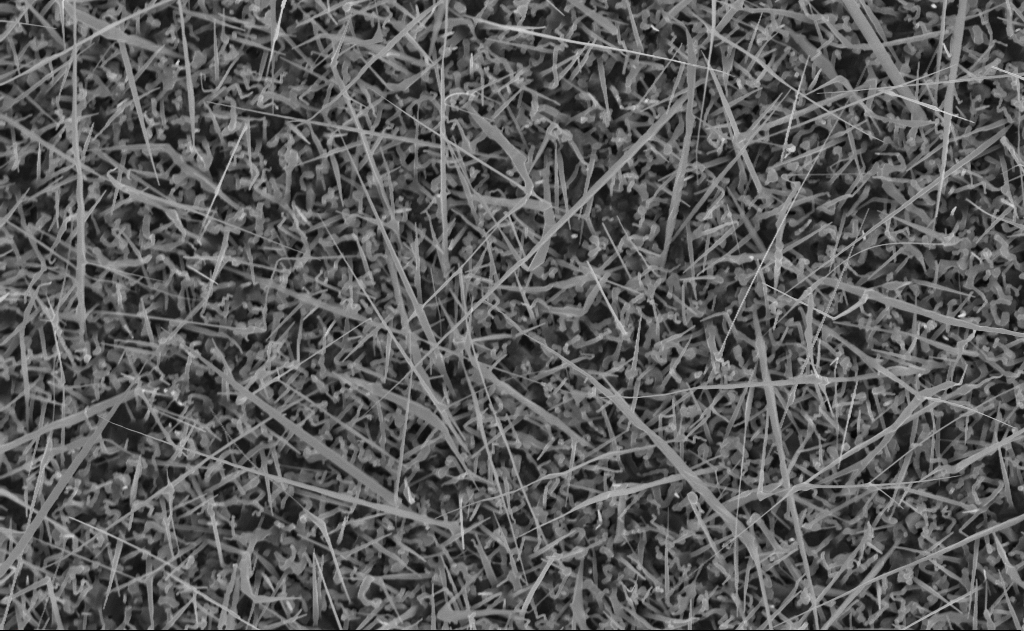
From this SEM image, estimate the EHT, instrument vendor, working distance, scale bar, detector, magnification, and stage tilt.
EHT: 10 kV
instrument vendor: Zeiss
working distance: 10 mm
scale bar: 2000 nm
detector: InLens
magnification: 20 K X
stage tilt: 0°